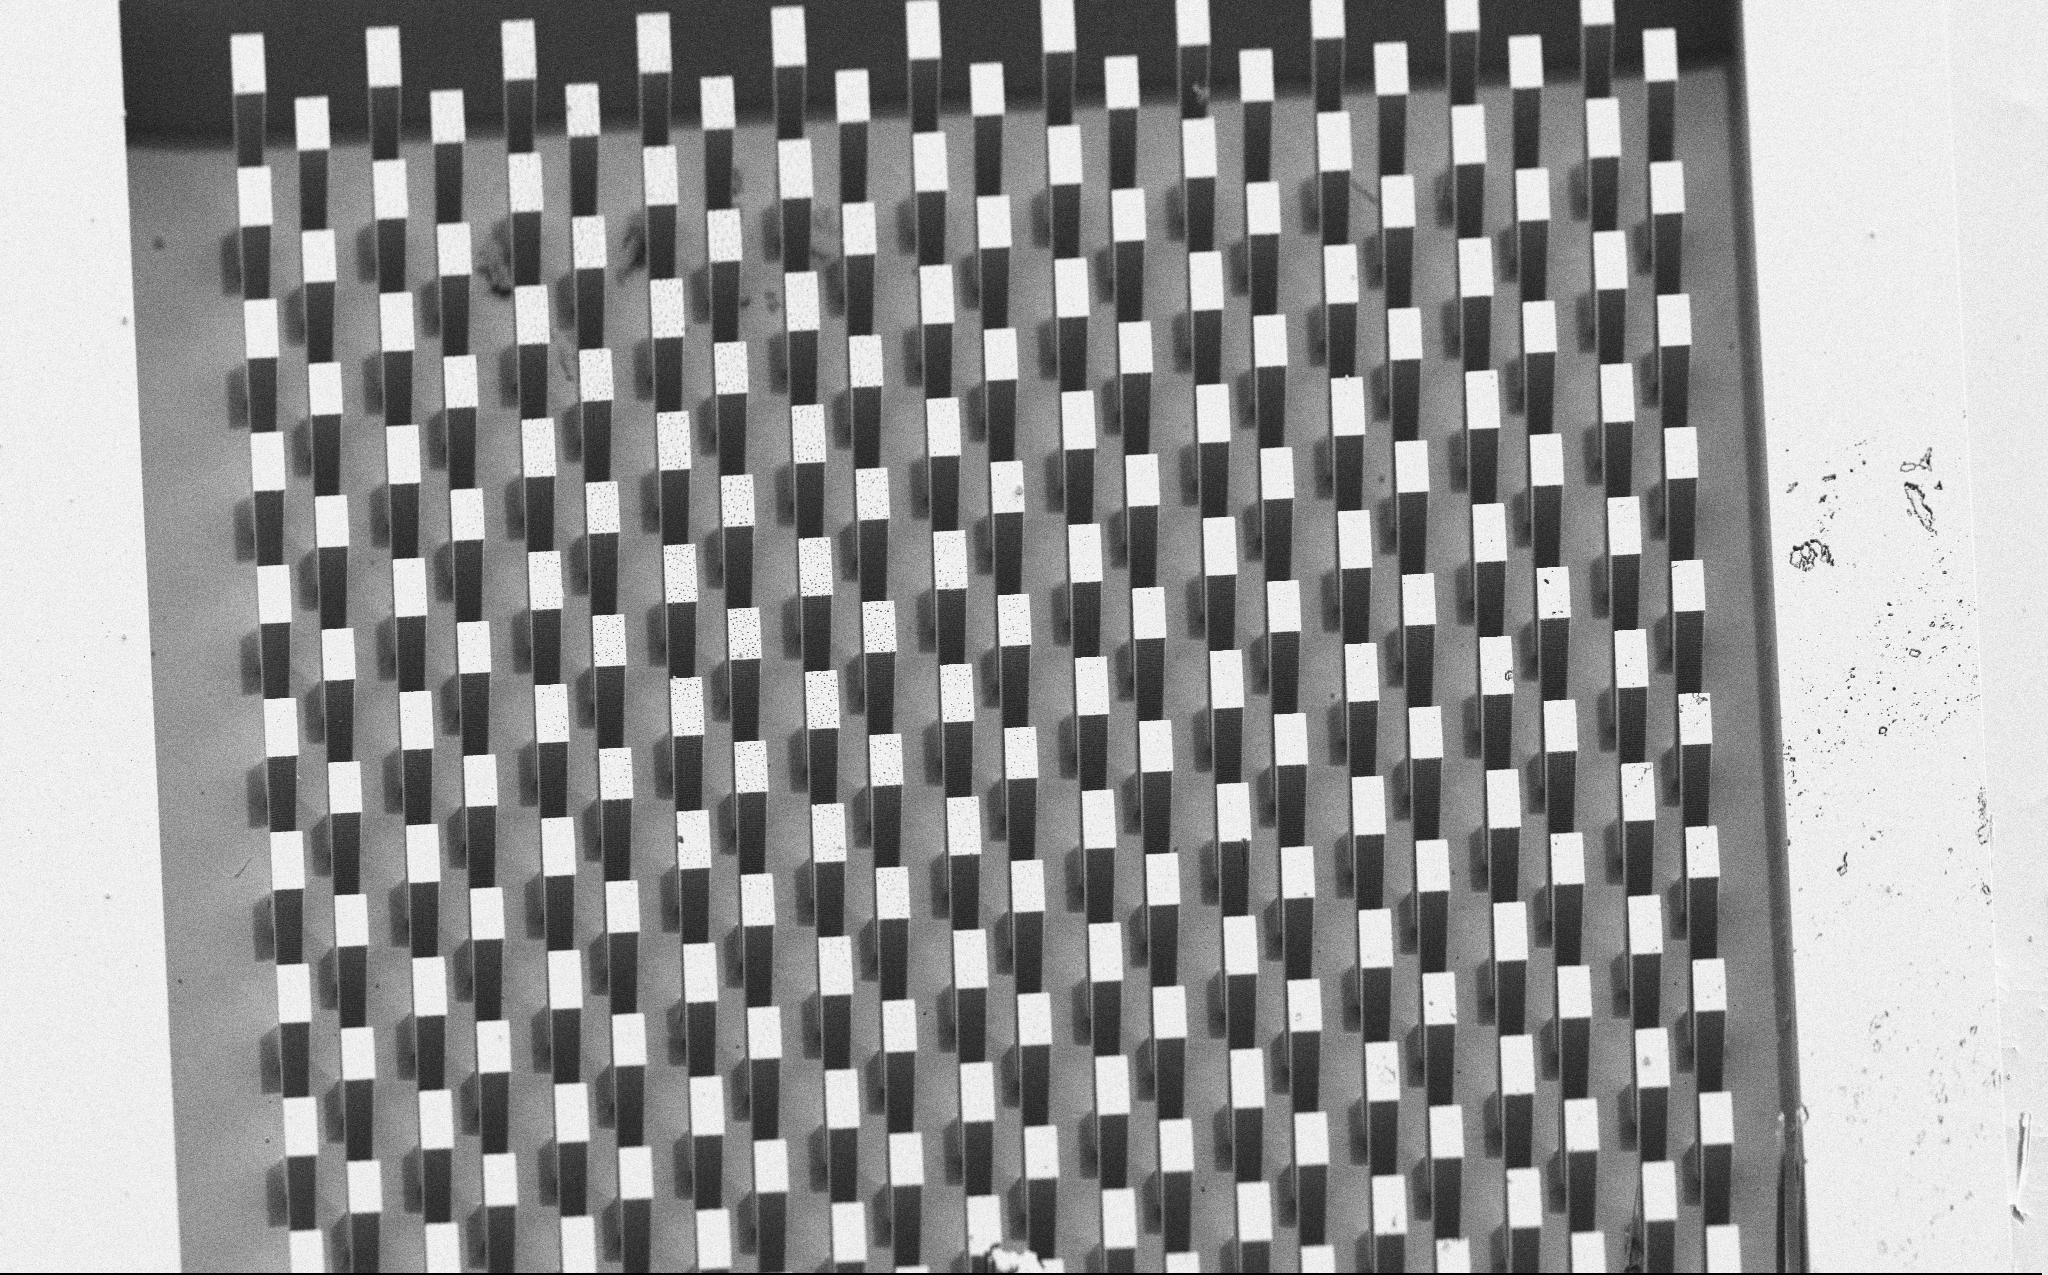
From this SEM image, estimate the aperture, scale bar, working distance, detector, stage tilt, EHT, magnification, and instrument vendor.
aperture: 30 µm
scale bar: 20000 nm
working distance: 8 mm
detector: SE2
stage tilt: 45°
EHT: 5 kV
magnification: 1.23 K X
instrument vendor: Zeiss